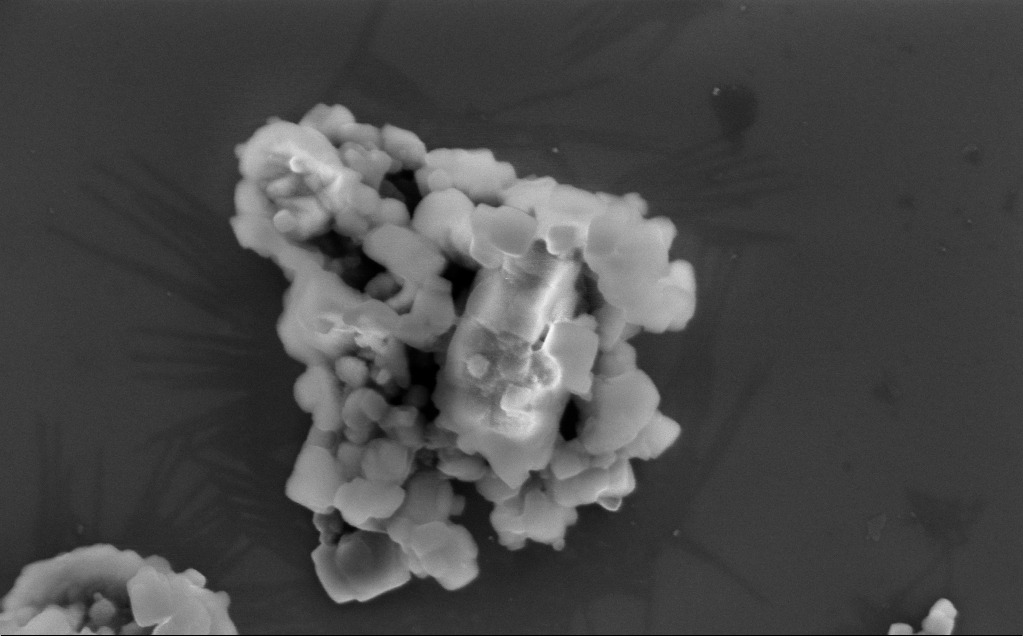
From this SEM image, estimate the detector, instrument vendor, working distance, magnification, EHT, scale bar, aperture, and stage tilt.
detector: InLens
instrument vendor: Zeiss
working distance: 3 mm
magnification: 124.47 K X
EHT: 3 kV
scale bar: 200 nm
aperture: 30 µm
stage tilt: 0°